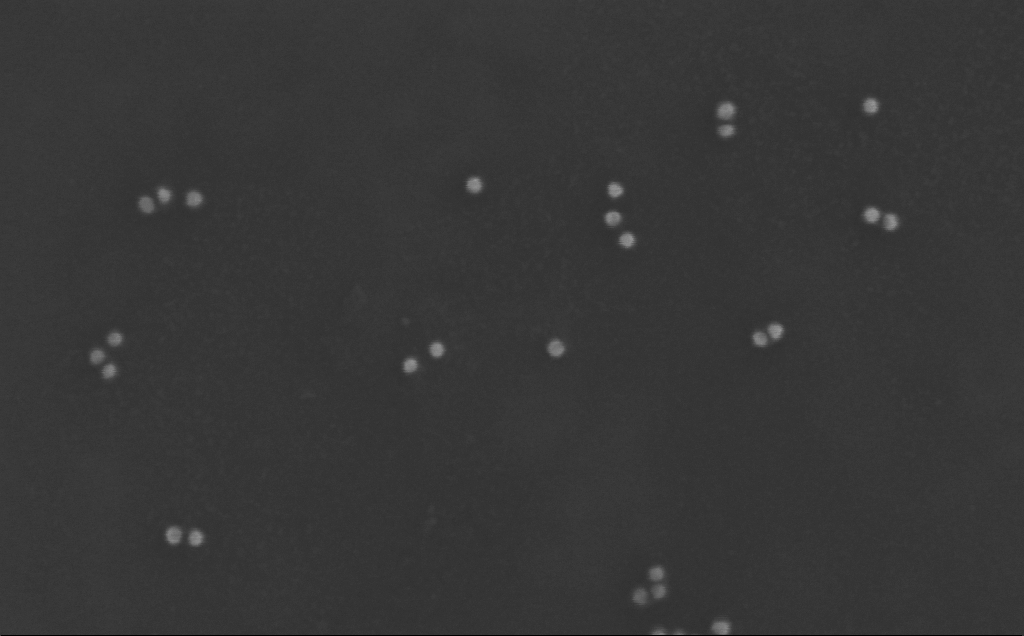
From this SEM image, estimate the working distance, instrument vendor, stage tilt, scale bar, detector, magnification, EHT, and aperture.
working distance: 3 mm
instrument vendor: Zeiss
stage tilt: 0°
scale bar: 100 nm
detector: InLens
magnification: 343.44 K X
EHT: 10 kV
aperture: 30 µm